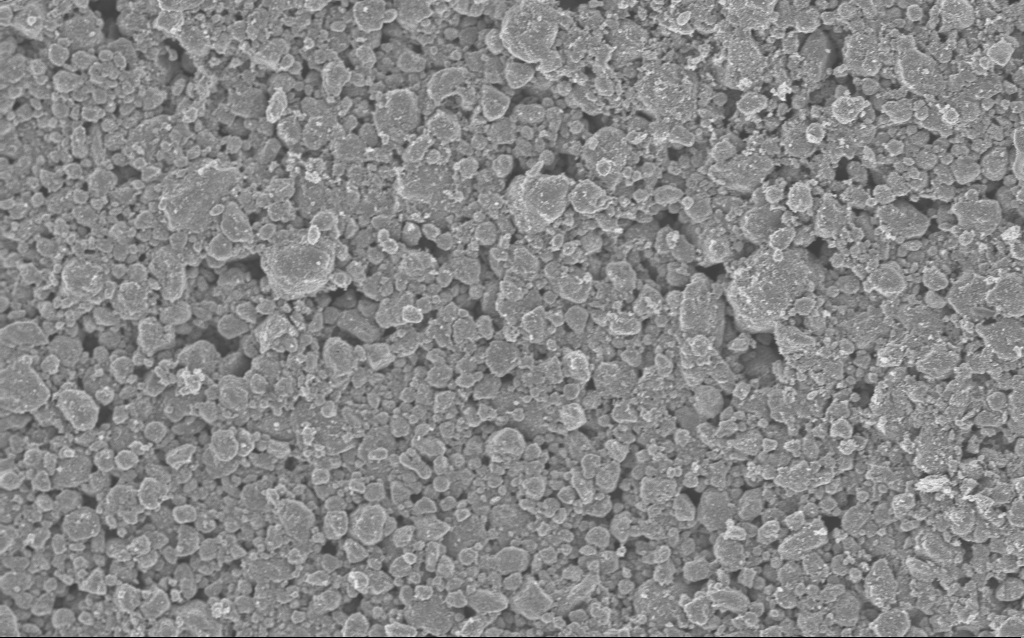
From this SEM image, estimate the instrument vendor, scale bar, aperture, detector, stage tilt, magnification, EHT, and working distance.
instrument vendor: Zeiss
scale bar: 10000 nm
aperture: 30 µm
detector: InLens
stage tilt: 0°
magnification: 2.74 K X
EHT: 5 kV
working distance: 4.7 mm